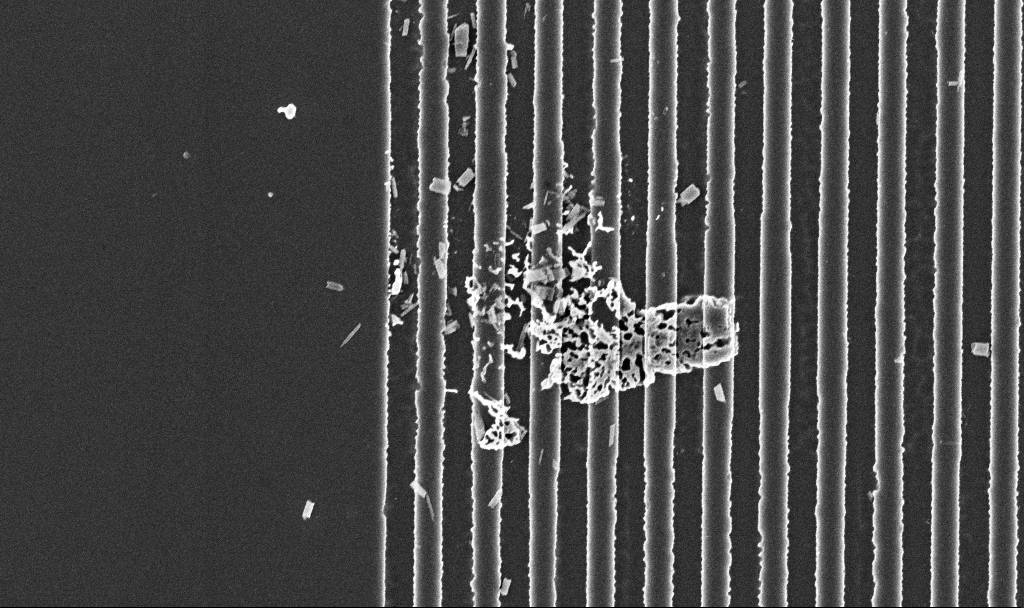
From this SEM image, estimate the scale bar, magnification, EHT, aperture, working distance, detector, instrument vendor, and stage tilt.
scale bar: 2000 nm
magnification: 32.38 K X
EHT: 3 kV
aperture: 30 µm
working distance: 2.9 mm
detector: InLens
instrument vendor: Zeiss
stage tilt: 0°